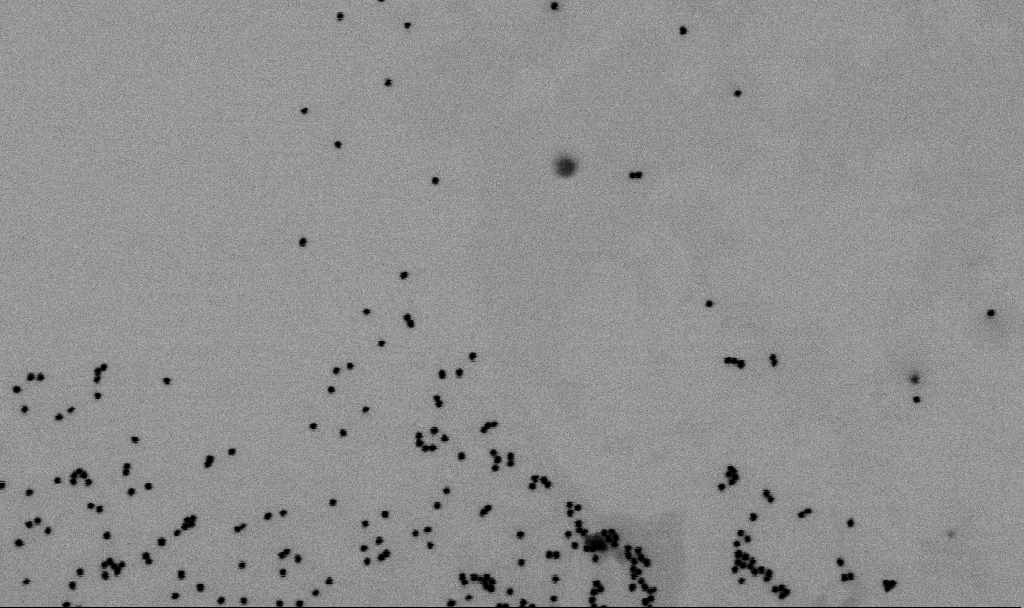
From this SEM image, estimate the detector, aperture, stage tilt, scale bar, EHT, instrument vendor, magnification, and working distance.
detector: SE2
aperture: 30 µm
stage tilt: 0°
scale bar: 200 nm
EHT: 2 kV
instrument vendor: Zeiss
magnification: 113.53 K X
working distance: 6.5 mm